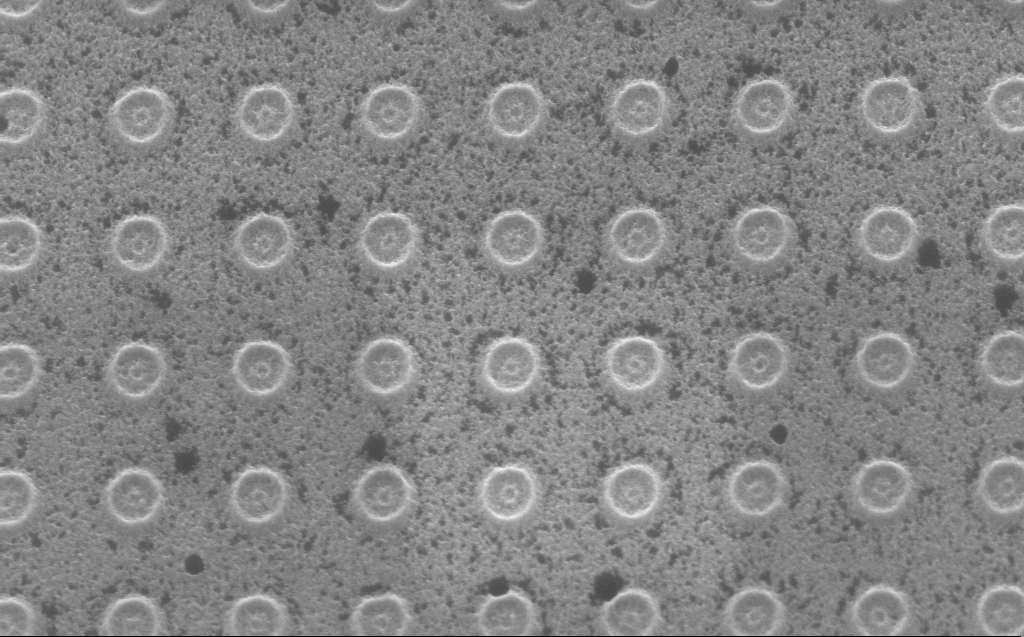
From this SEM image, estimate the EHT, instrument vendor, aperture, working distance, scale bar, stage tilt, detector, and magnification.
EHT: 5 kV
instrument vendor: Zeiss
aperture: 30 µm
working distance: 4 mm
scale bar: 1000 nm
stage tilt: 30°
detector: InLens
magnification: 57.97 K X